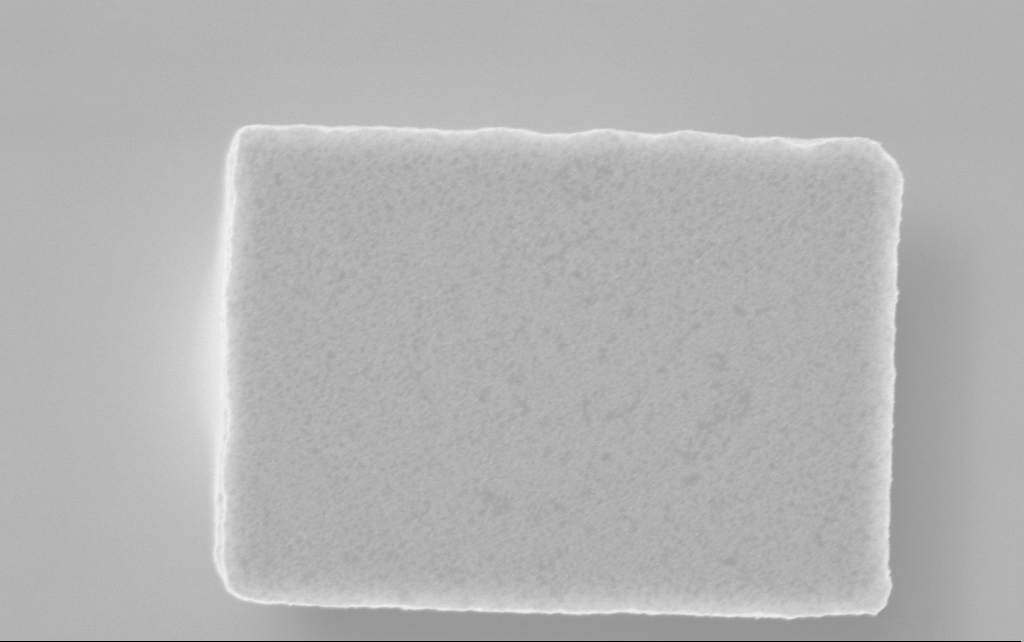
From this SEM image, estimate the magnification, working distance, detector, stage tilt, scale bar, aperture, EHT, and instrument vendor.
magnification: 81.8 K X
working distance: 2.5 mm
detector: InLens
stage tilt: -0°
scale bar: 200 nm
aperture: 30 µm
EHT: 3 kV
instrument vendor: Zeiss